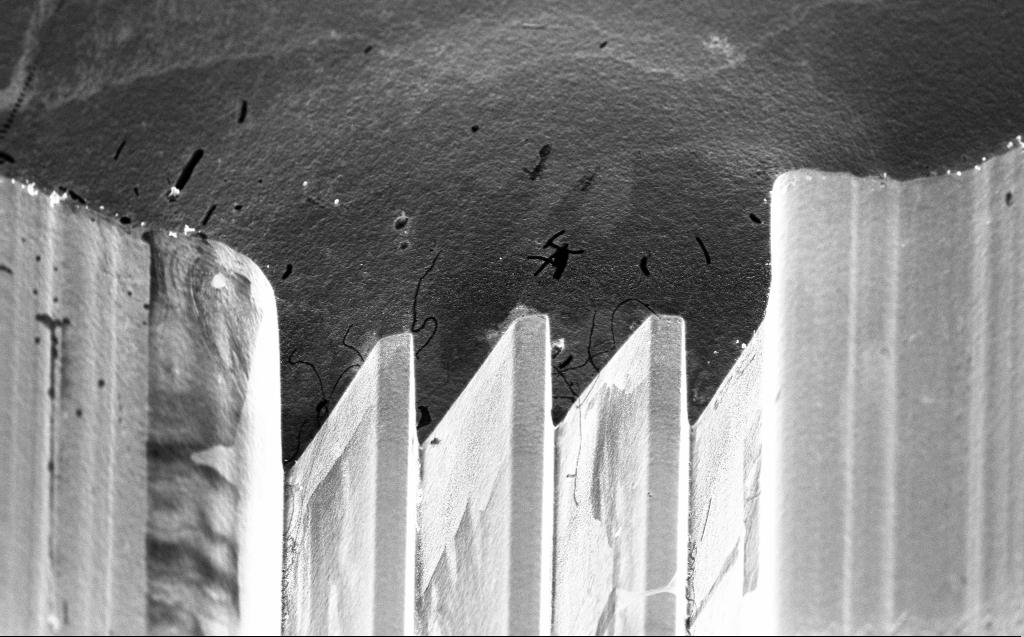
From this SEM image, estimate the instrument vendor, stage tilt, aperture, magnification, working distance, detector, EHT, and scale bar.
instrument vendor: Zeiss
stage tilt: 45°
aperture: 30 µm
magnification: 4.37 K X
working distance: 3 mm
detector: InLens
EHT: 10 kV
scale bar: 10000 nm